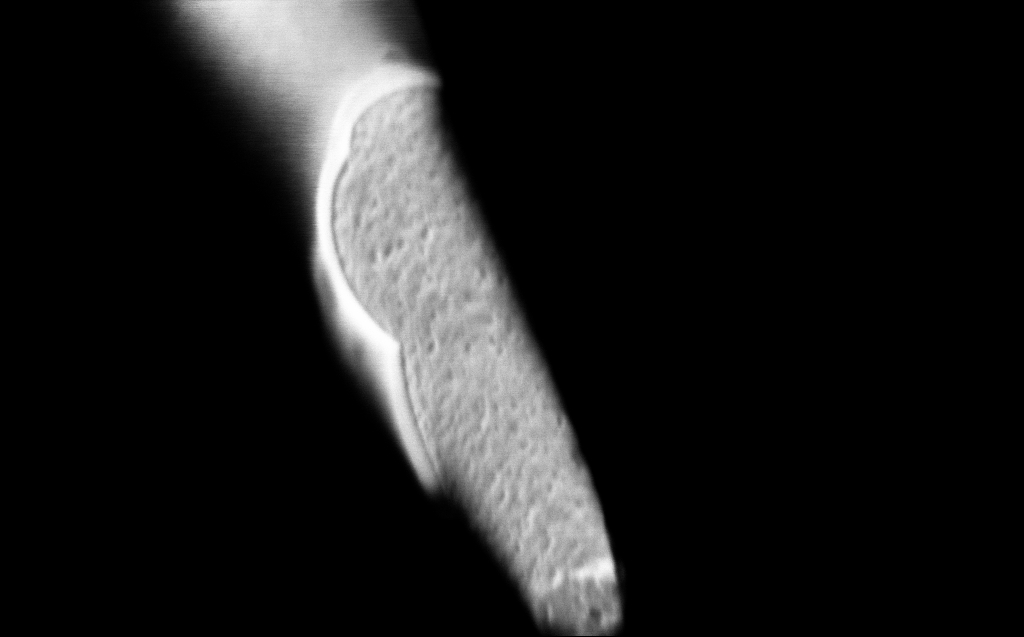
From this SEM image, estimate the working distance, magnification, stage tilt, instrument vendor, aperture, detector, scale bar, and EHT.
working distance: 4 mm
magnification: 100 K X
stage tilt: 45°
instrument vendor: Zeiss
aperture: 30 µm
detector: InLens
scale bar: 200 nm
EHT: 0.8 kV